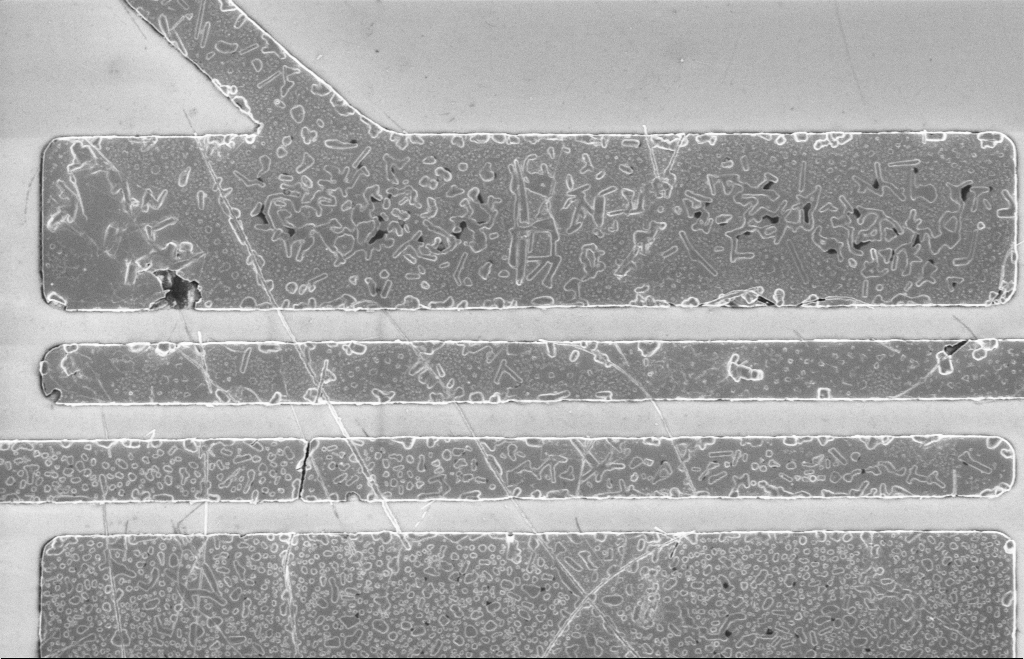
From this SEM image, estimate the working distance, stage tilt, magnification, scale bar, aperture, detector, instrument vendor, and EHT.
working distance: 8 mm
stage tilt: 0°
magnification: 5.91 K X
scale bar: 2000 nm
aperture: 20 µm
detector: InLens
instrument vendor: Zeiss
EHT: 5 kV